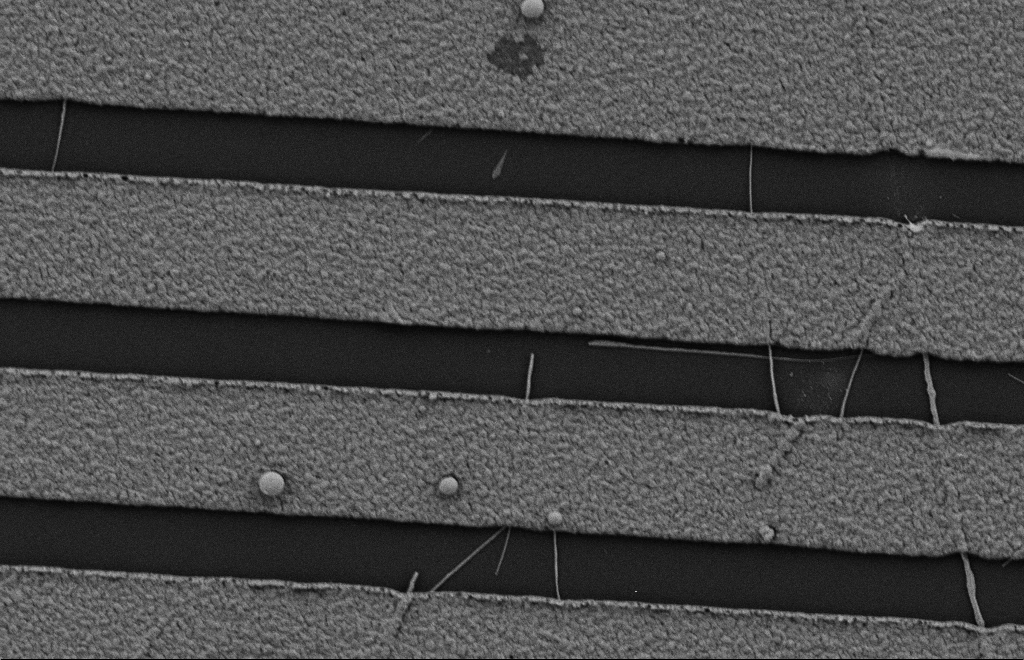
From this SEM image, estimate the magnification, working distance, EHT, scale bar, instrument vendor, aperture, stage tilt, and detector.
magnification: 18.28 K X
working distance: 10 mm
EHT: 2 kV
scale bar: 2000 nm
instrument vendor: Zeiss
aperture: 20 µm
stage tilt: -0.3°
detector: SE2